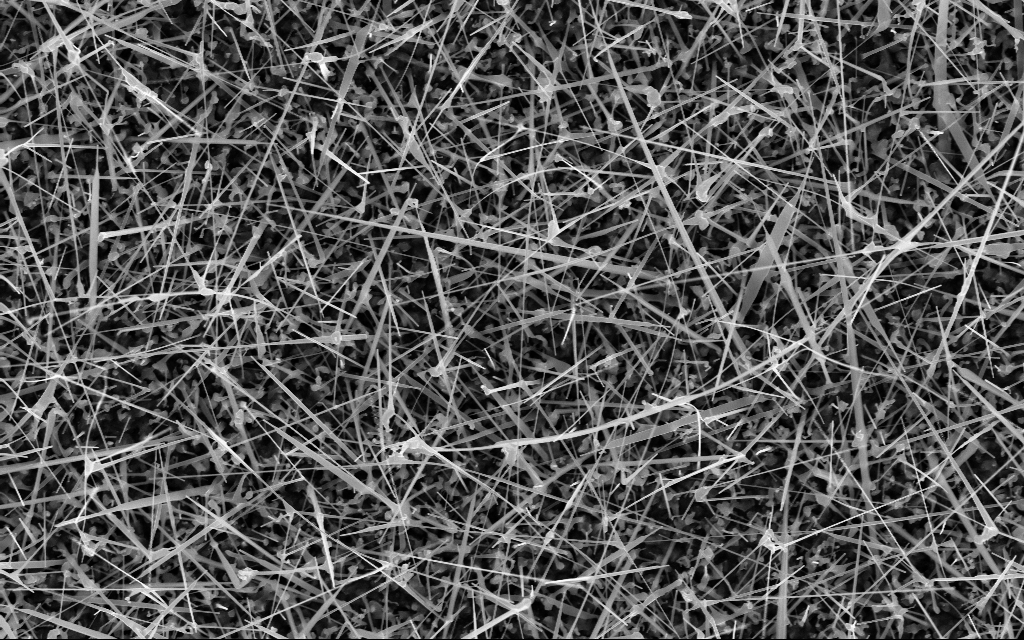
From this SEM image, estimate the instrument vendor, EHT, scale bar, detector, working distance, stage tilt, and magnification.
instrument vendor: Zeiss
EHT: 10 kV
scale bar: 2000 nm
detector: InLens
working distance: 7 mm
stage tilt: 0°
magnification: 20 K X